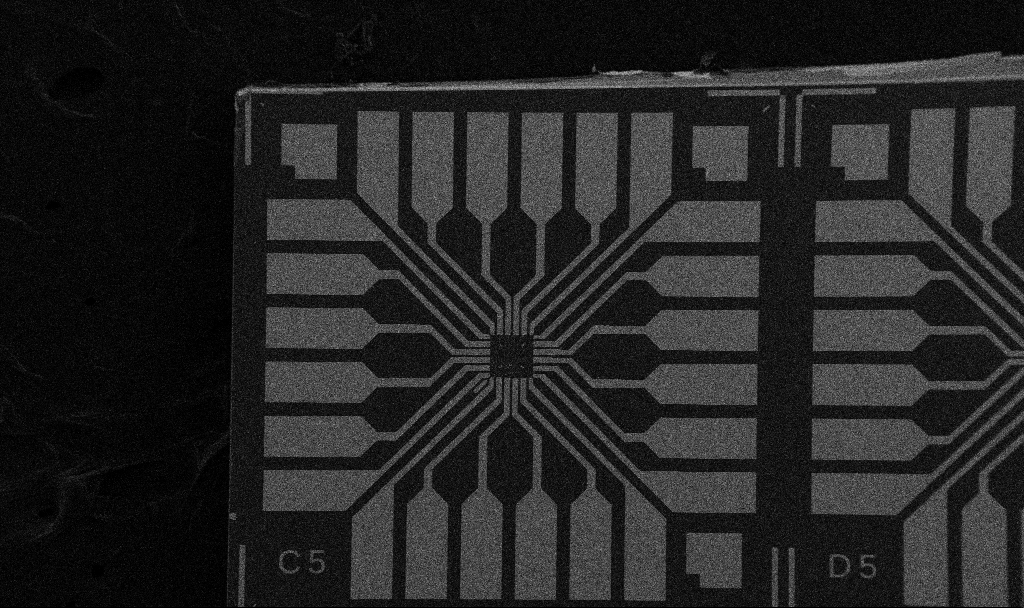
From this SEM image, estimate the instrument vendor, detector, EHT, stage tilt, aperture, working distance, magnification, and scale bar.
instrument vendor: Zeiss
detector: SE2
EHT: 5 kV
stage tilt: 0°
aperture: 30 µm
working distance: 10.7 mm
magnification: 0.1 K X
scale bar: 200000 nm